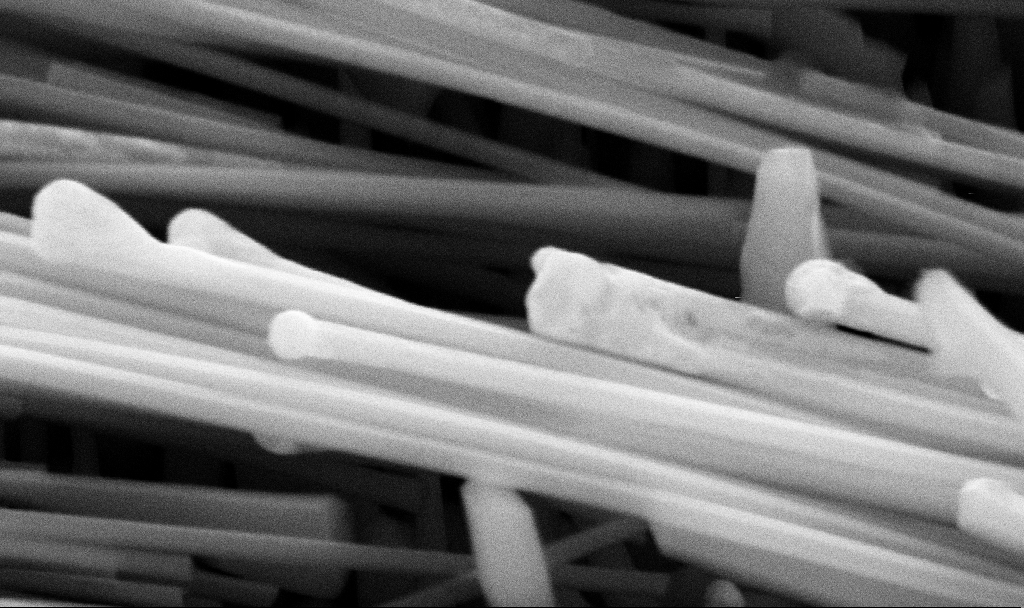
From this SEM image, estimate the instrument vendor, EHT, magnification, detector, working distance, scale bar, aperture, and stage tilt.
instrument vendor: Zeiss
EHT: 10 kV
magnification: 108.61 K X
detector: SE2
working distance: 11.2 mm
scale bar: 200 nm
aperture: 30 µm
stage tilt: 45°